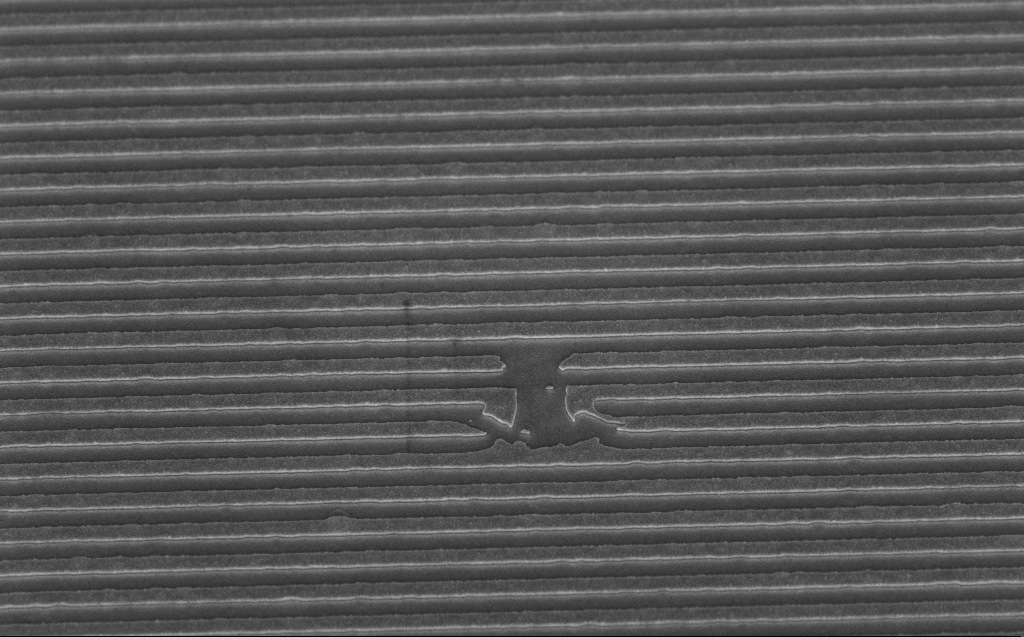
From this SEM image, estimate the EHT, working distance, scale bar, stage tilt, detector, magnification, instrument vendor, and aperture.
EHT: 5 kV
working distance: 5 mm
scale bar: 1000 nm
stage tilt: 45°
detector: InLens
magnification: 24.54 K X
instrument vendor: Zeiss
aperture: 30 µm